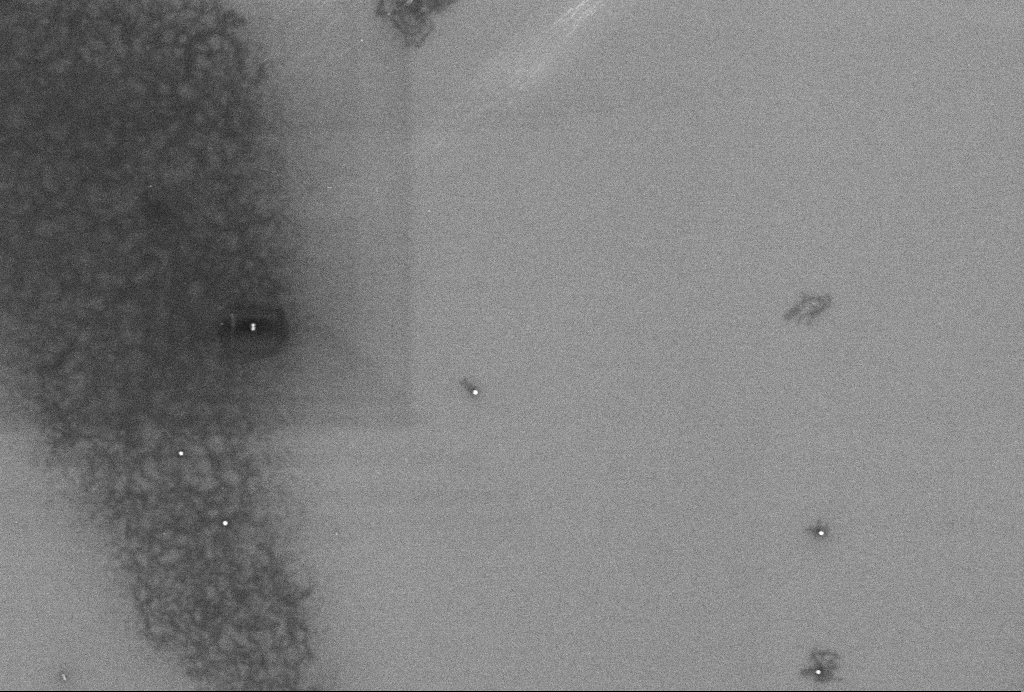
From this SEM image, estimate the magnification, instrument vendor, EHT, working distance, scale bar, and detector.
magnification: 56.73 K X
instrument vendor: Zeiss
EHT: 2 kV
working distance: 3.3 mm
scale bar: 1000 nm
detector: InLens